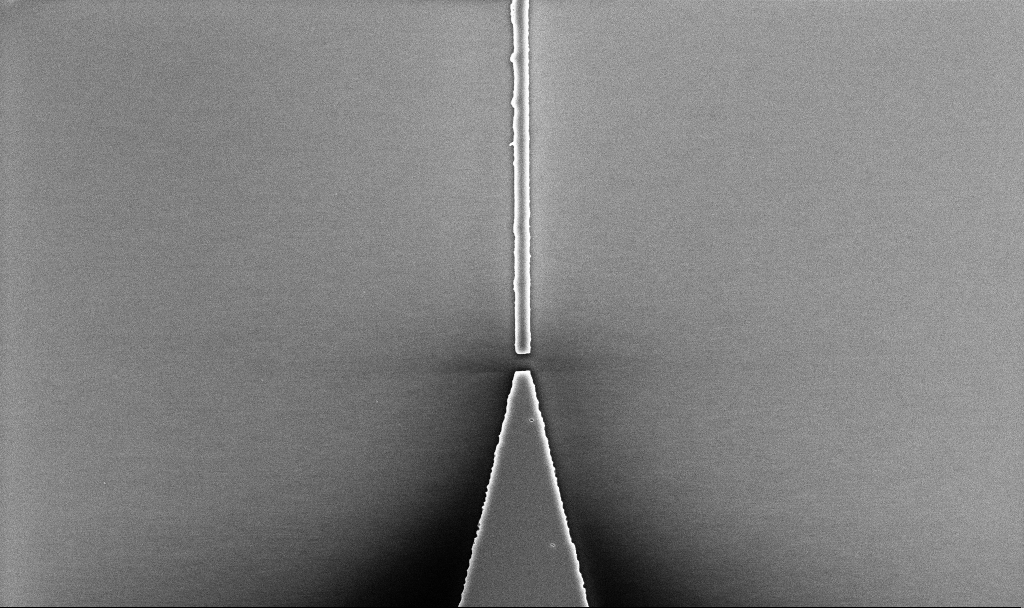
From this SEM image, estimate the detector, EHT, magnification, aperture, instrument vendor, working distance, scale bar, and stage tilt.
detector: InLens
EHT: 5 kV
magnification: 11 K X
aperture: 30 µm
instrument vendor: Zeiss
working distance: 5.2 mm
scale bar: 2000 nm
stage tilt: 0°